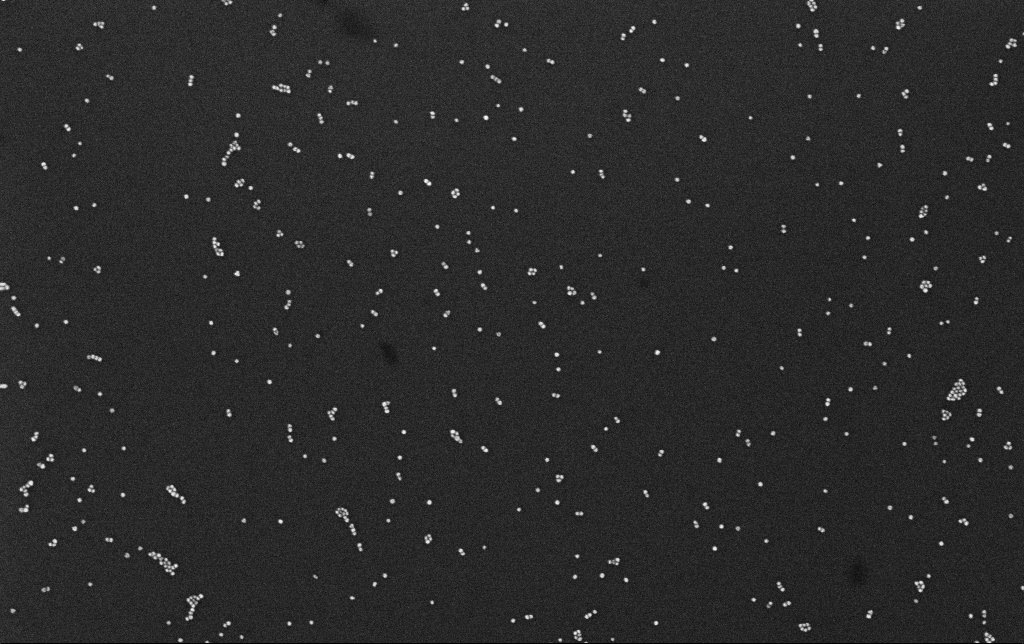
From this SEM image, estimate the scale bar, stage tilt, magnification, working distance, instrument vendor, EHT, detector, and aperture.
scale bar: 200 nm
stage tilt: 0°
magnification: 100 K X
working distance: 3.1 mm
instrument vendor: Zeiss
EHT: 10 kV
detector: InLens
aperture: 30 µm